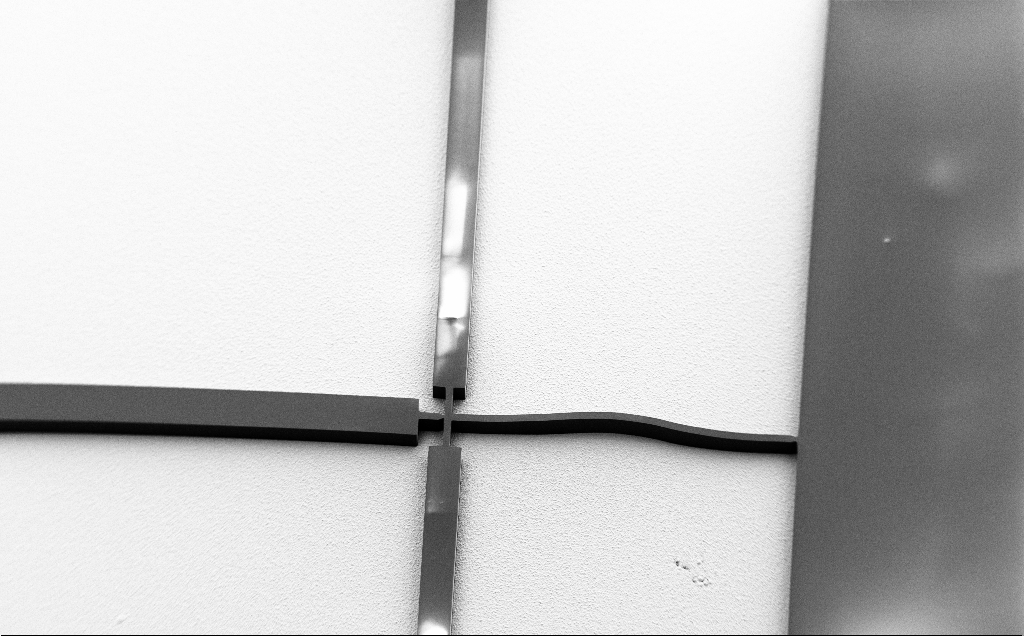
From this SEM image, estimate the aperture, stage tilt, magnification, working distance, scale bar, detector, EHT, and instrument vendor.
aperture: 30 µm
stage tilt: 37.9°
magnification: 0.245 K X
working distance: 8 mm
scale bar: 100000 nm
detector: SE2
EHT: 1.5 kV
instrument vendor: Zeiss